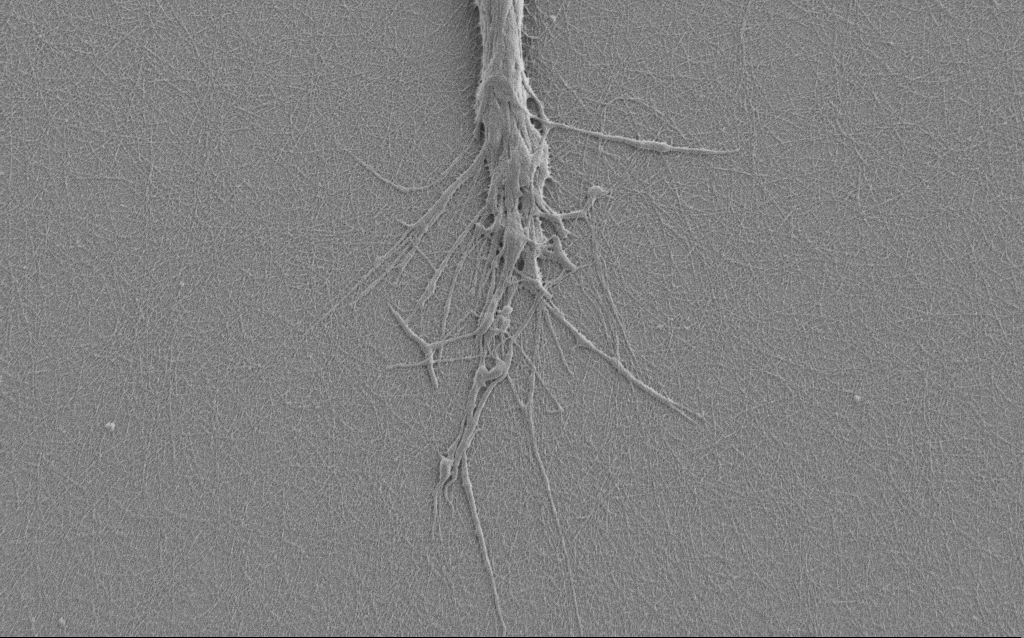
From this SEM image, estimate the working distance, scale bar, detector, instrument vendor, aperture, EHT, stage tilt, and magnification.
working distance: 6 mm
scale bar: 2000 nm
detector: SE2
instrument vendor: Zeiss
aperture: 30 µm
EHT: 1 kV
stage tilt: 0°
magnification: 7.5 K X